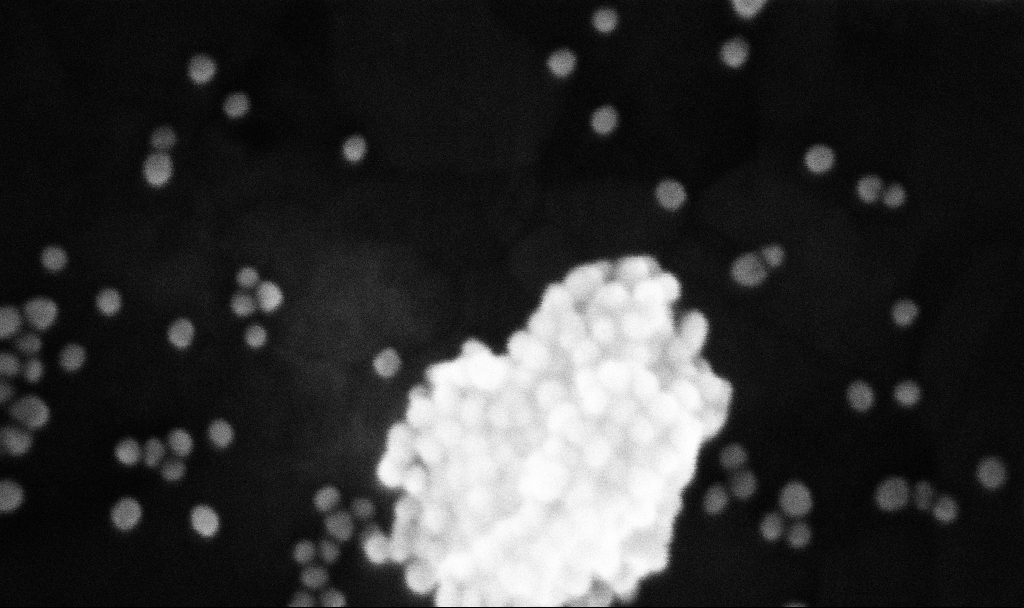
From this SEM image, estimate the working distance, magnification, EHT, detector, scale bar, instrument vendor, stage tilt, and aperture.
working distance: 3.7 mm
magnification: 608.57 K X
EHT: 10 kV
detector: InLens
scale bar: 100 nm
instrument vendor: Zeiss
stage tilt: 0°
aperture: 30 µm